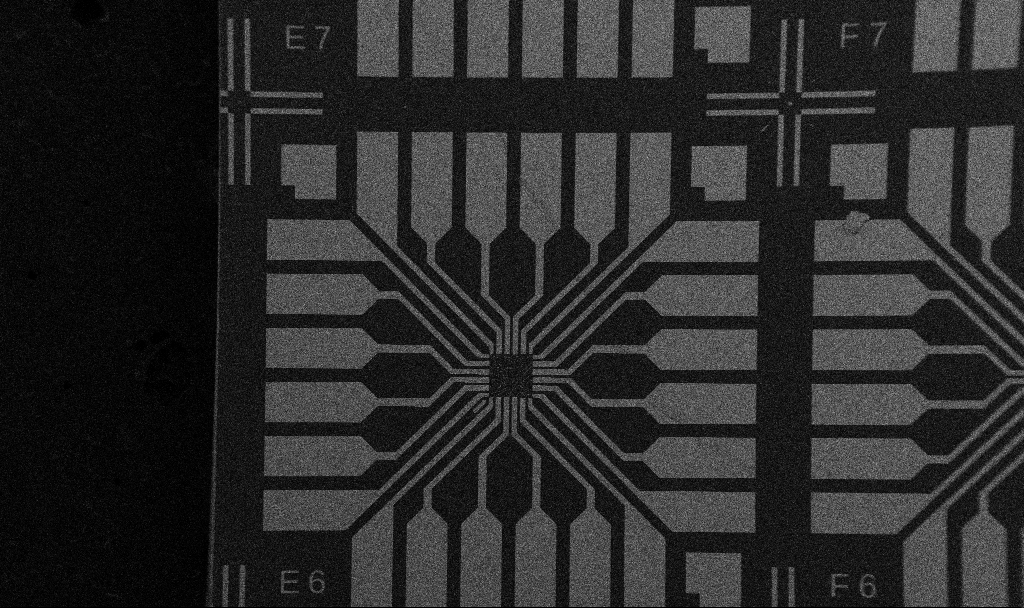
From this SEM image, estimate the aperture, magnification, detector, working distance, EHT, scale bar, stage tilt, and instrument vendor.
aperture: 30 µm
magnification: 0.1 K X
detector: SE2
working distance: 10.7 mm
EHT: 5 kV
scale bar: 200000 nm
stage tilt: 0°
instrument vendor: Zeiss